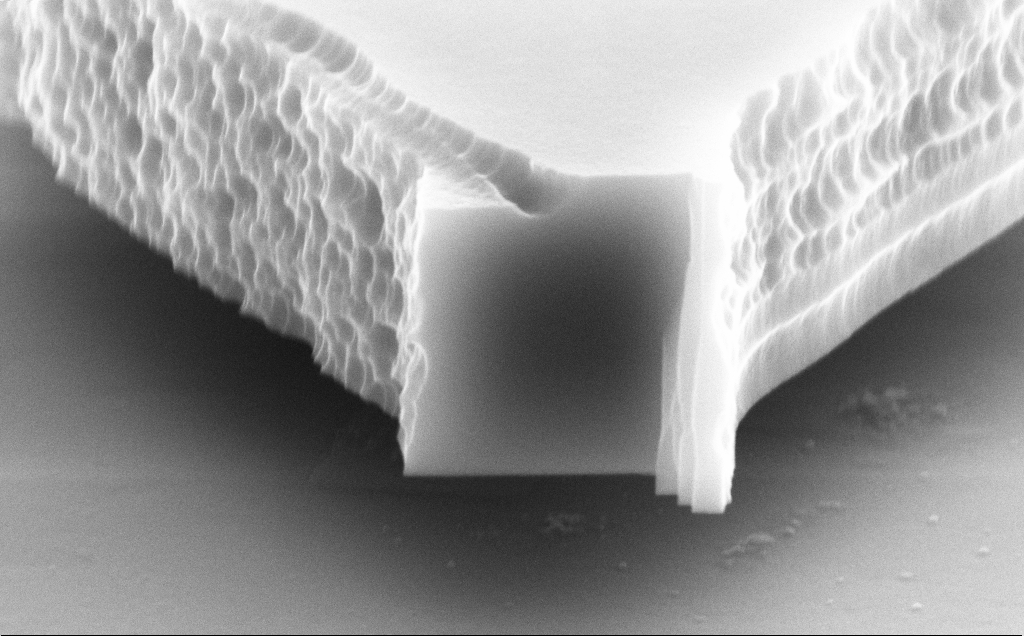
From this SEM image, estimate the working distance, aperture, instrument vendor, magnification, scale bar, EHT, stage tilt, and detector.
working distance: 9 mm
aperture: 30 µm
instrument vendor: Zeiss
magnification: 47.87 K X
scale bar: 1000 nm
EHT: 10 kV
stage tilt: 70°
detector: SE2